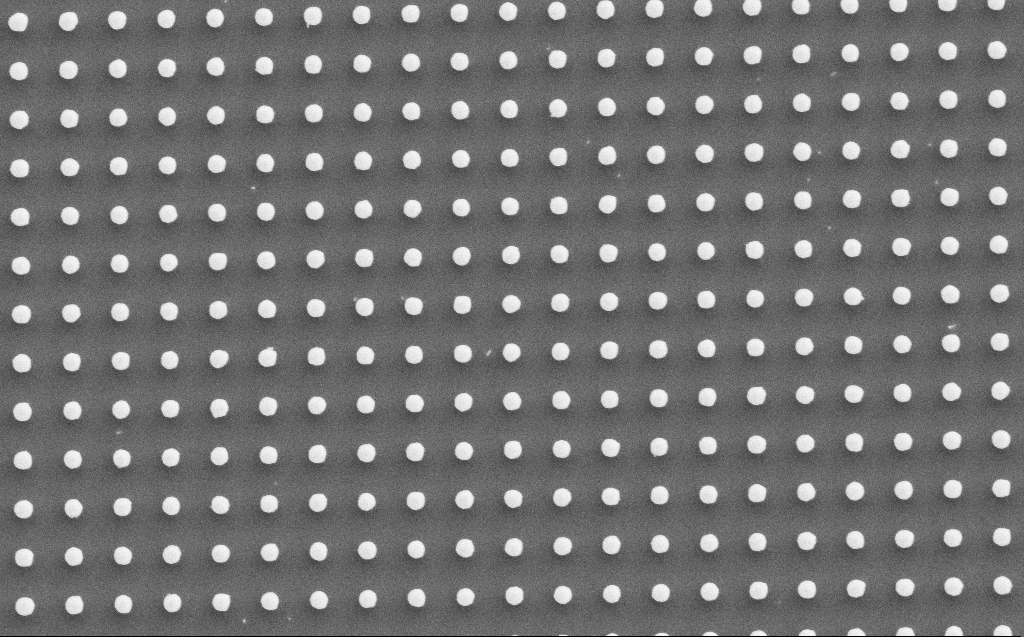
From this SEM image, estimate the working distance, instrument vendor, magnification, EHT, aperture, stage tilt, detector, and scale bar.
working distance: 5 mm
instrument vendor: Zeiss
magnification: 17.17 K X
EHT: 5 kV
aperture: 30 µm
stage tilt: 0°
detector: SE2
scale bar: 1000 nm